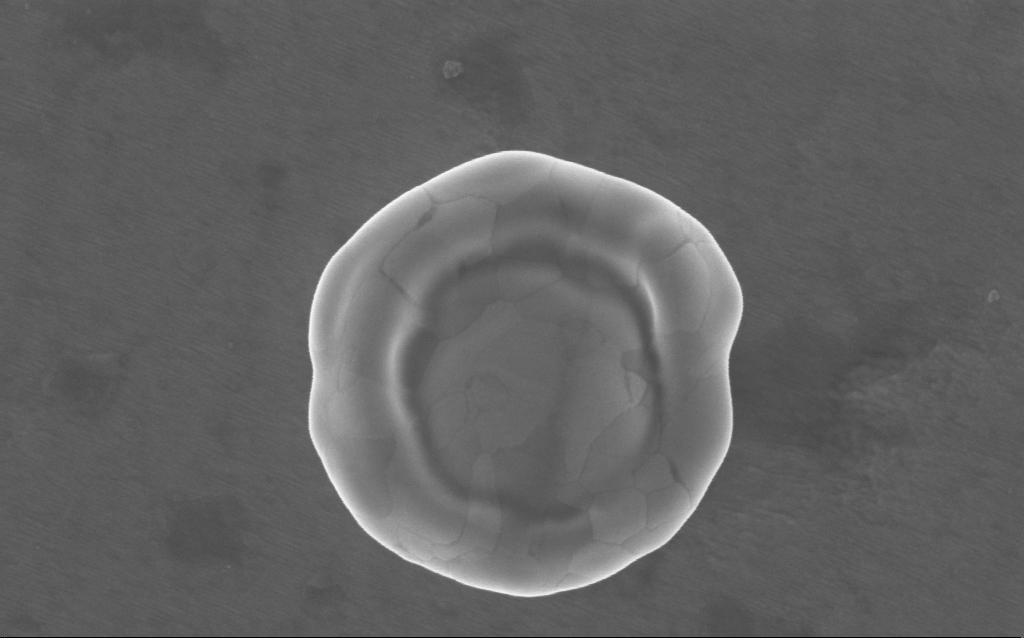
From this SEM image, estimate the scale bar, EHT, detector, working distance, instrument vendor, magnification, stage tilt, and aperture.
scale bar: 100 nm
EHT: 5 kV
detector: InLens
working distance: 3 mm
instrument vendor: Zeiss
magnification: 121 K X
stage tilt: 0°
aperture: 30 µm